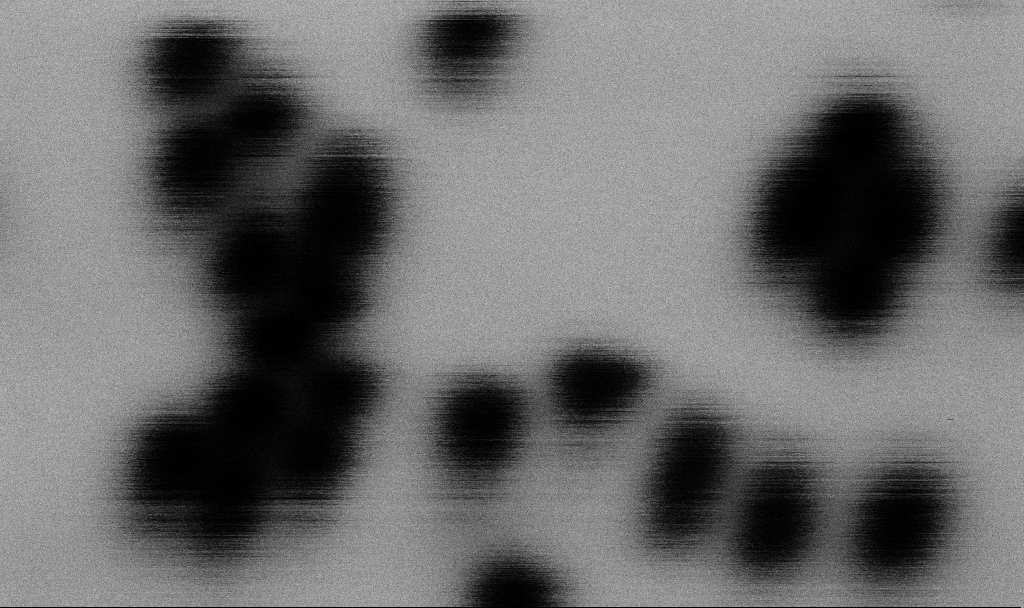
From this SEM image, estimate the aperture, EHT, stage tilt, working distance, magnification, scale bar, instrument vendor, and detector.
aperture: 30 µm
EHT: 2 kV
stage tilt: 0°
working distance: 6.5 mm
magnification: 1376.59 K X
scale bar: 20 nm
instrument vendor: Zeiss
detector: SE2